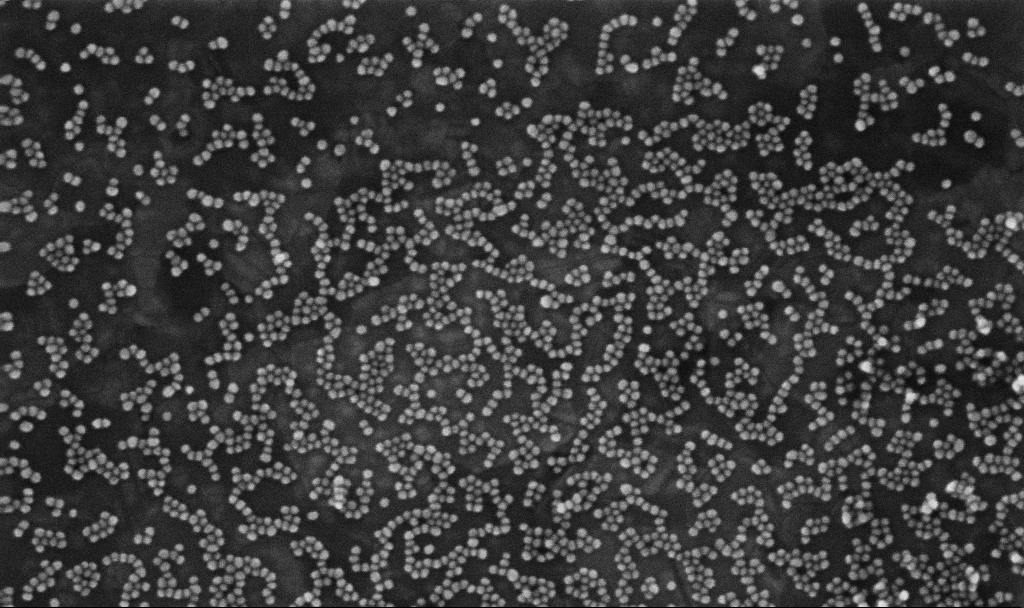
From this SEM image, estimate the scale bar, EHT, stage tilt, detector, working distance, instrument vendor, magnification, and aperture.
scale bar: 200 nm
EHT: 10 kV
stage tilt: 0°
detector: InLens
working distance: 3.8 mm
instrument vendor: Zeiss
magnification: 200 K X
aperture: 30 µm